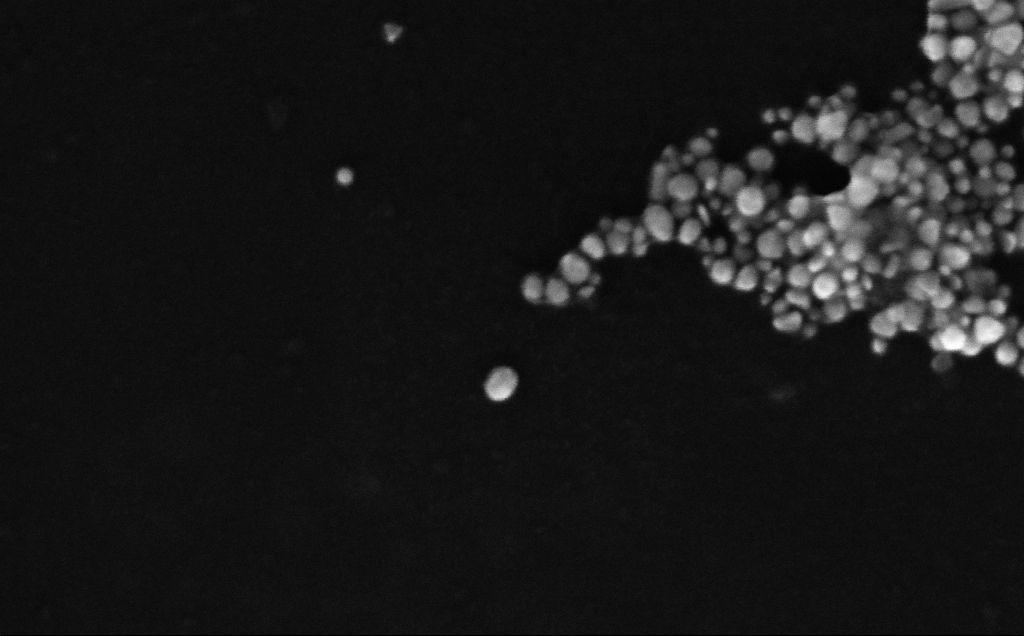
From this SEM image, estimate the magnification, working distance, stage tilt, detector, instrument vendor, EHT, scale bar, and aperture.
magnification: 431.93 K X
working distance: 4 mm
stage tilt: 0°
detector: InLens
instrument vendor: Zeiss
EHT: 10 kV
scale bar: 100 nm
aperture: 30 µm